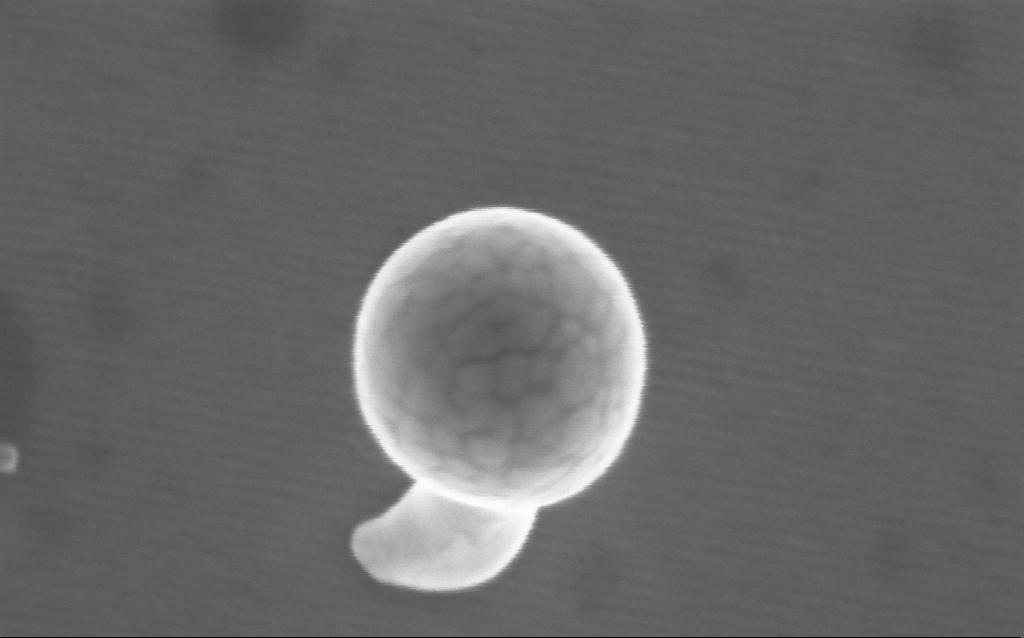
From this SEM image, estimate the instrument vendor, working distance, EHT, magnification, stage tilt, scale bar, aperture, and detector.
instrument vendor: Zeiss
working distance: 4 mm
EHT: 5 kV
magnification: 366.3 K X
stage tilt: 0°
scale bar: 100 nm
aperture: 30 µm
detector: InLens